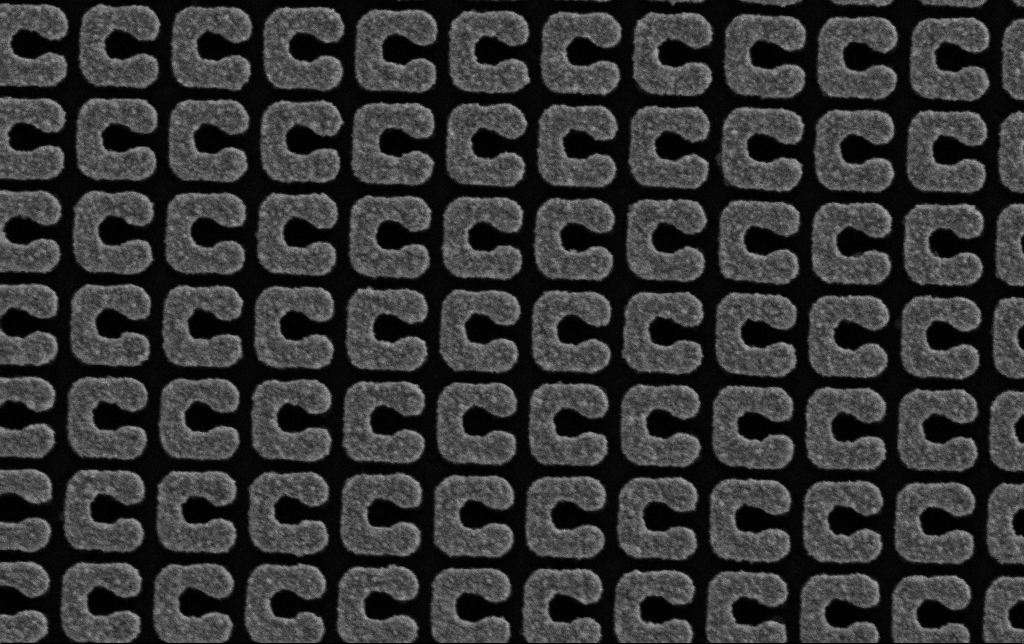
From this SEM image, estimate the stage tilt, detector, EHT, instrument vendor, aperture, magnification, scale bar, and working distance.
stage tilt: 0°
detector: SE2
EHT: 3 kV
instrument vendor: Zeiss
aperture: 30 µm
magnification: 73.57 K X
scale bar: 200 nm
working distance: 5.1 mm